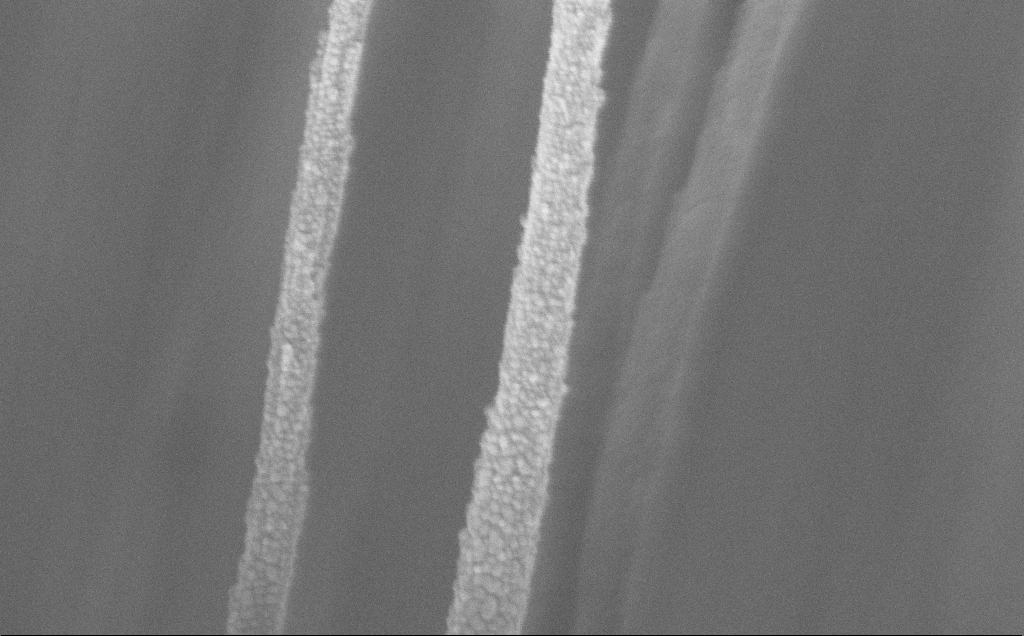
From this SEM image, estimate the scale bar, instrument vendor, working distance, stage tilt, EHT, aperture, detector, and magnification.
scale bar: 200 nm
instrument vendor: Zeiss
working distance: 11 mm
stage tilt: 0°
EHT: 5 kV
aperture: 30 µm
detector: InLens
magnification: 136.92 K X